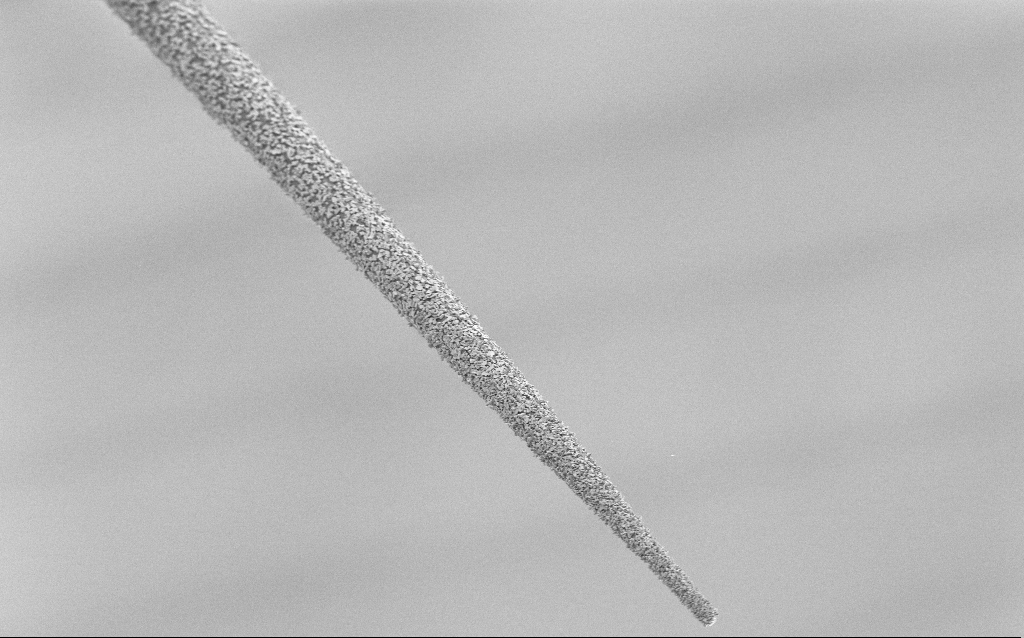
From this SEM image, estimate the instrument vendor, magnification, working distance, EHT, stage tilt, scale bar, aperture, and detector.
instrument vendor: Zeiss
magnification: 1 K X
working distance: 7.8 mm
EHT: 1.5 kV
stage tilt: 45°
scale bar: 20000 nm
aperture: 30 µm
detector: SE2